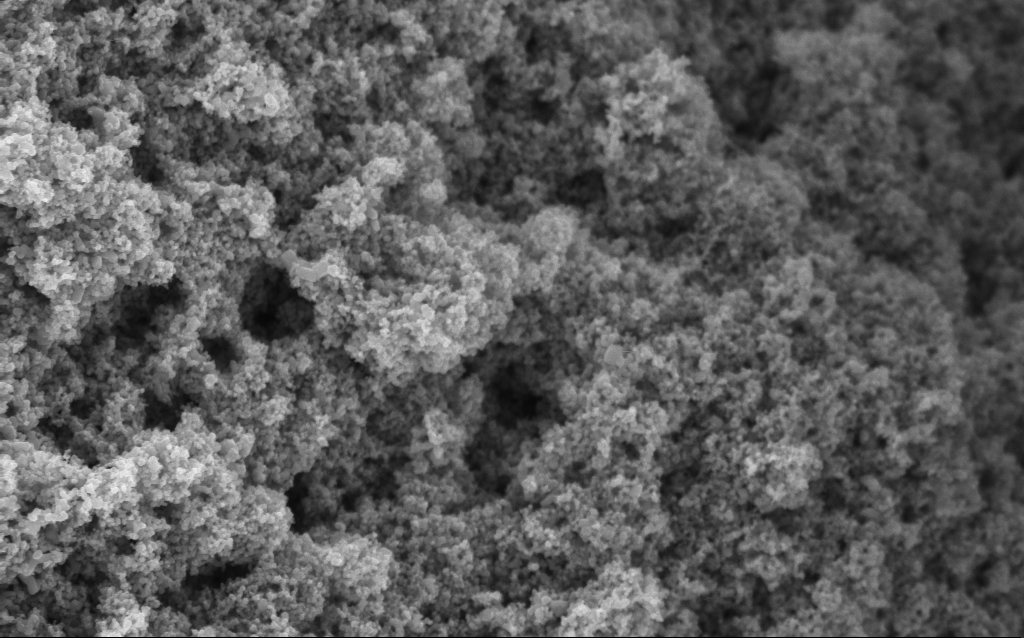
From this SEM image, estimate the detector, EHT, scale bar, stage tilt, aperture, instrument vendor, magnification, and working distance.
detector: InLens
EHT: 5 kV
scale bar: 1000 nm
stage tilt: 0°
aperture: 30 µm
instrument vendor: Zeiss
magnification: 68.65 K X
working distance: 4.5 mm